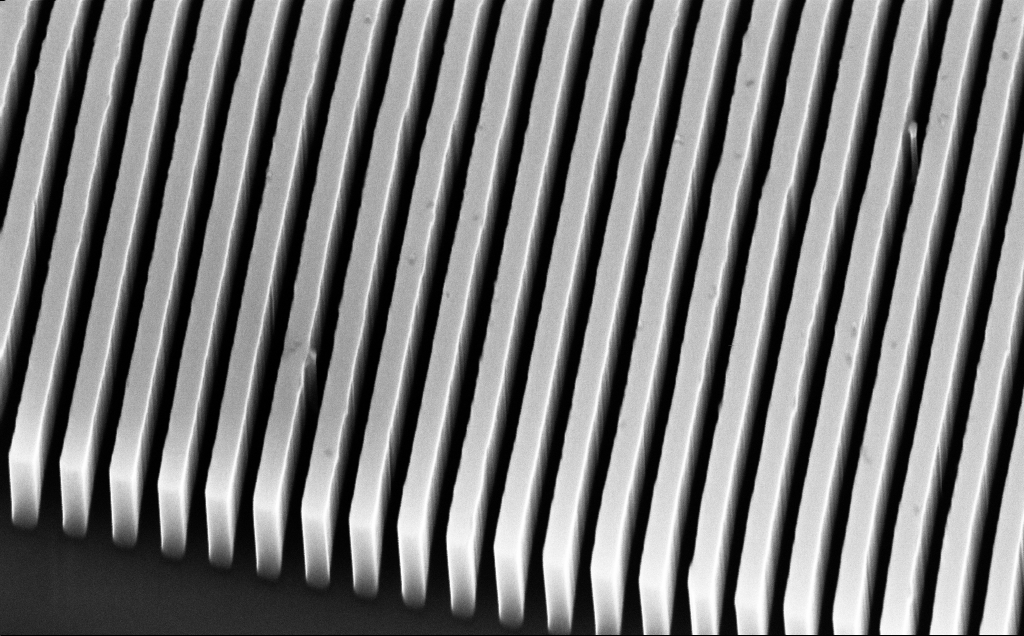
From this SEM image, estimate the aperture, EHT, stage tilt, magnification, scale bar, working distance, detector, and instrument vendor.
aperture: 30 µm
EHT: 10 kV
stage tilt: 45°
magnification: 60.29 K X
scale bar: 1000 nm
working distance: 6 mm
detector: InLens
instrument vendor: Zeiss